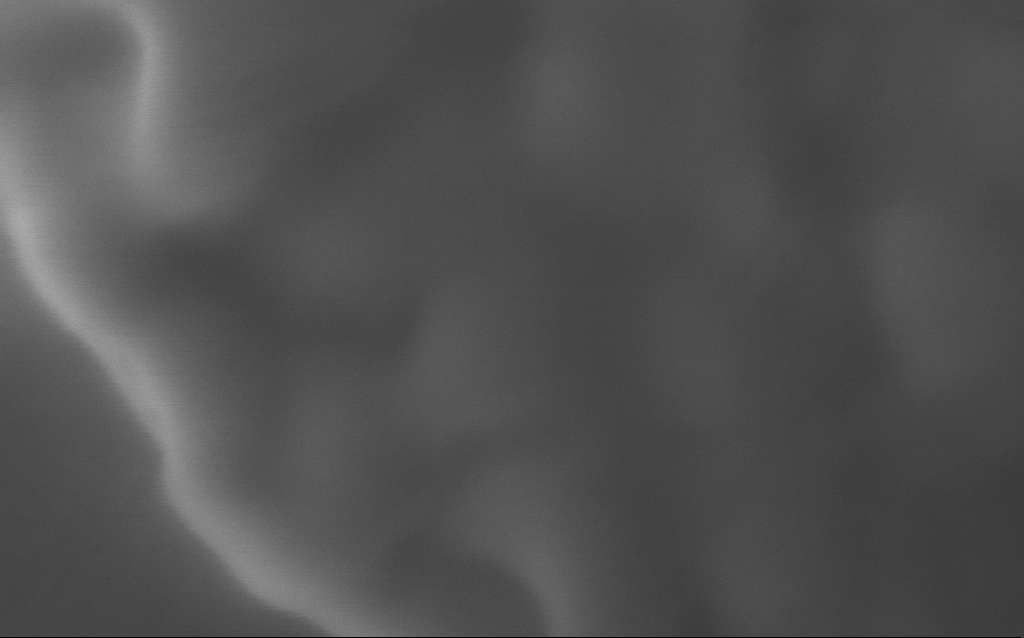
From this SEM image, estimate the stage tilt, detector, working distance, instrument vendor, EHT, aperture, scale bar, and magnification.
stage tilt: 0°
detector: InLens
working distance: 2 mm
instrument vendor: Zeiss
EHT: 5 kV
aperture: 30 µm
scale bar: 100 nm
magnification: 680.59 K X